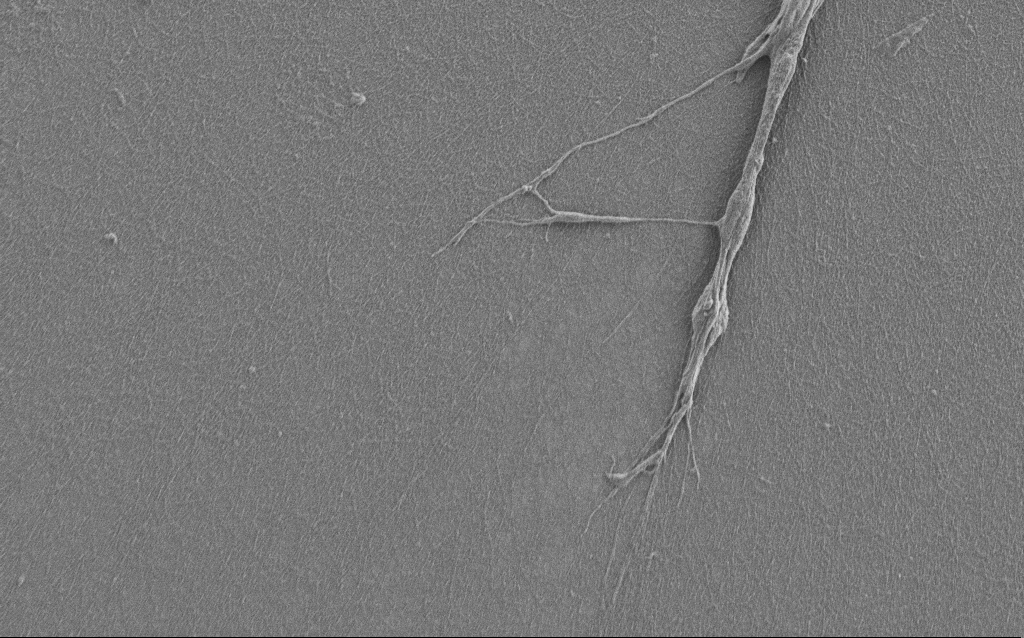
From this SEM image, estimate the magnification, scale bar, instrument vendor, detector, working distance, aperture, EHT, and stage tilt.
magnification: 7.5 K X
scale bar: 2000 nm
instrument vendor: Zeiss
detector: SE2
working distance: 6 mm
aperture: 30 µm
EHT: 1 kV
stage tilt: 0°